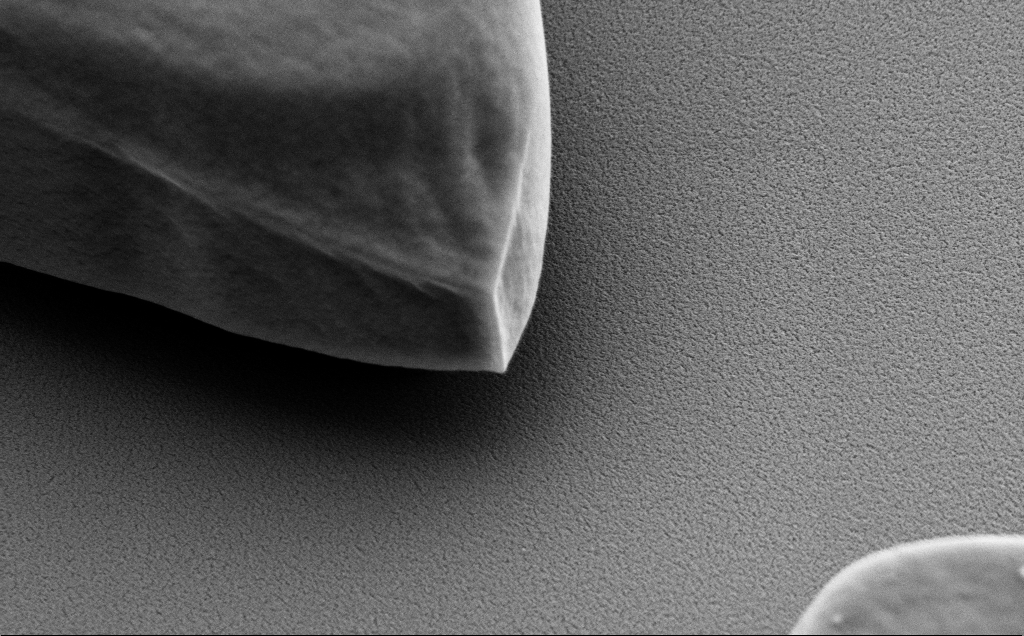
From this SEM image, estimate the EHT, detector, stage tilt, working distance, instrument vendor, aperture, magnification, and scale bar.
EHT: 5 kV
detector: SE2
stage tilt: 40°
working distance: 9 mm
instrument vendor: Zeiss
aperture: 30 µm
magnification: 27.77 K X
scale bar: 2000 nm